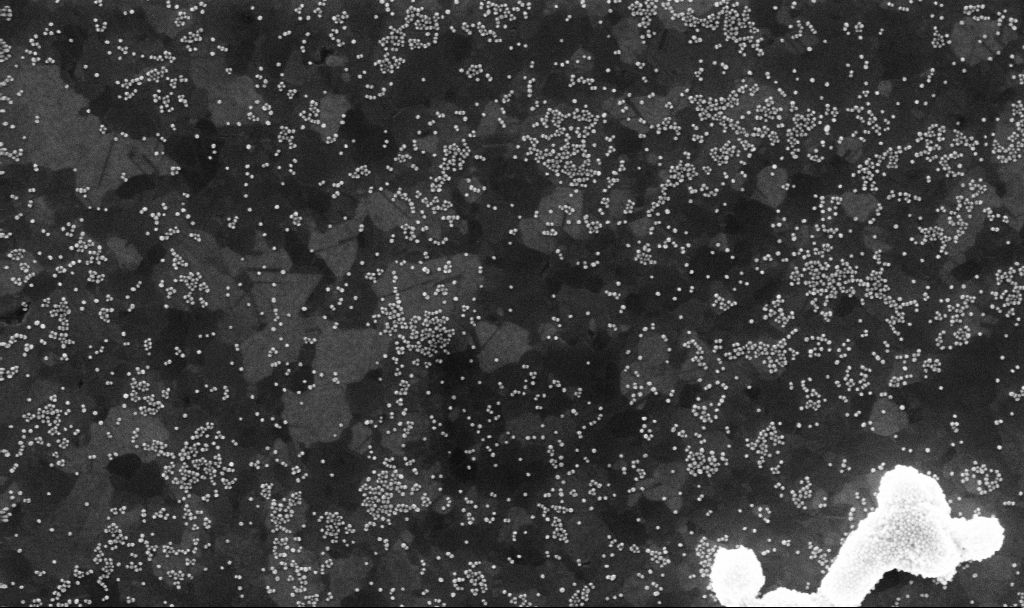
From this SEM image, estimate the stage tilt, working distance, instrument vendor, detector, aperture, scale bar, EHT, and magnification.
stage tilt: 0°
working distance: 3.8 mm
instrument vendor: Zeiss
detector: InLens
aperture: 30 µm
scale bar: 200 nm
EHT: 10 kV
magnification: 80 K X